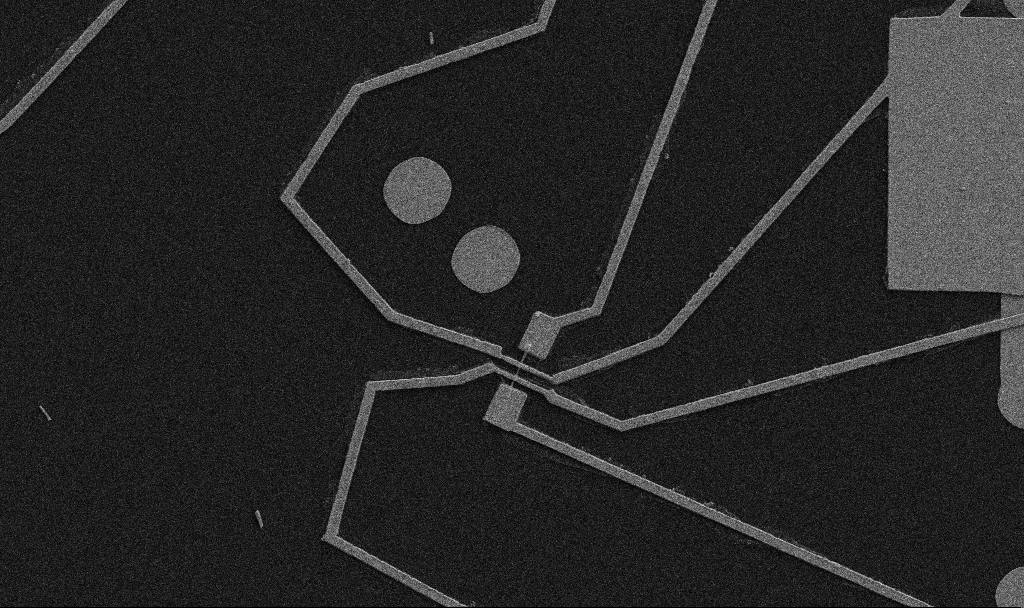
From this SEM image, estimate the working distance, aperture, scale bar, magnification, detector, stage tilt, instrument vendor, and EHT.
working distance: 10.7 mm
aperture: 30 µm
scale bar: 10000 nm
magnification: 5 K X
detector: SE2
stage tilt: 0°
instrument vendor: Zeiss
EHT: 5 kV